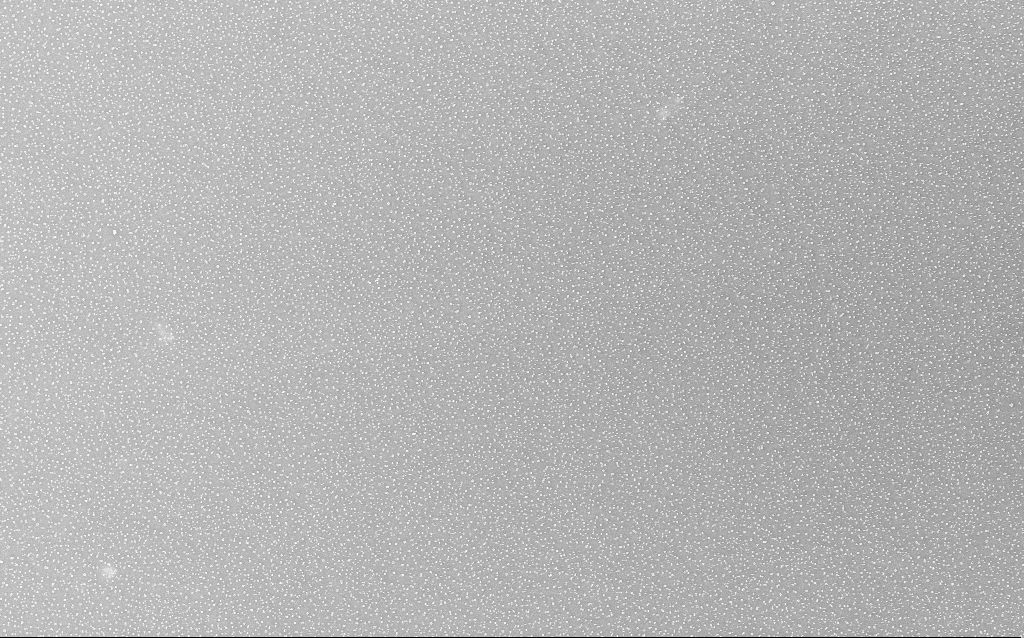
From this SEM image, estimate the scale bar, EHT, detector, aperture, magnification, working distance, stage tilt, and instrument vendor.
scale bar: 20000 nm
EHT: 20 kV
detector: InLens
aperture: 30 µm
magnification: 1 K X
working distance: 3.4 mm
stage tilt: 0°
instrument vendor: Zeiss